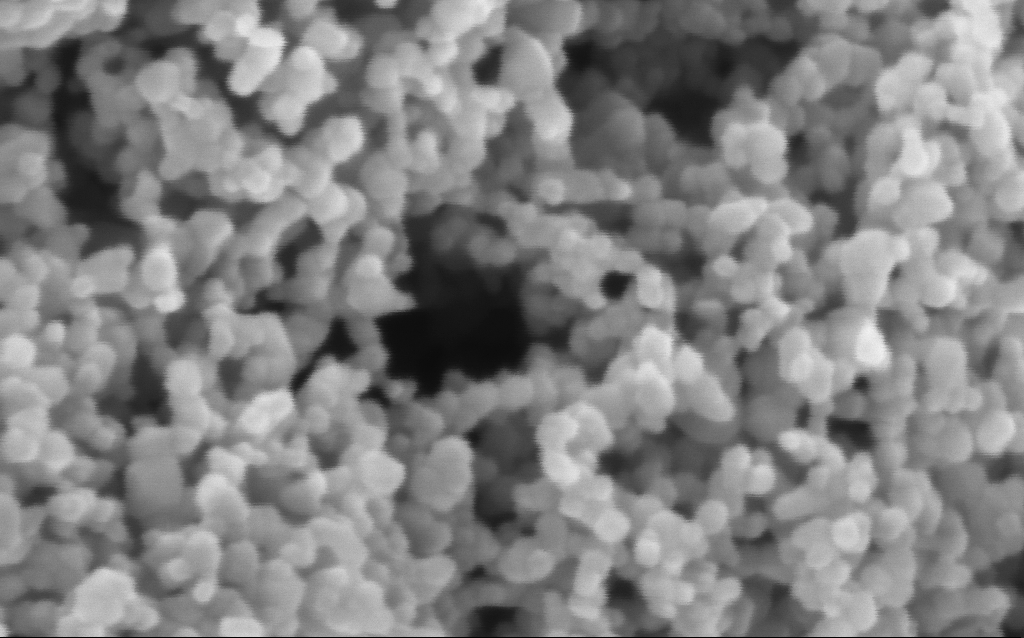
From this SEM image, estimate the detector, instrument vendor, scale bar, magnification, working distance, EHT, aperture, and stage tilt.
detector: InLens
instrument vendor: Zeiss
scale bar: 100 nm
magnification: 416 K X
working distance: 7.5 mm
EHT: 3 kV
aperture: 30 µm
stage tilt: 0°